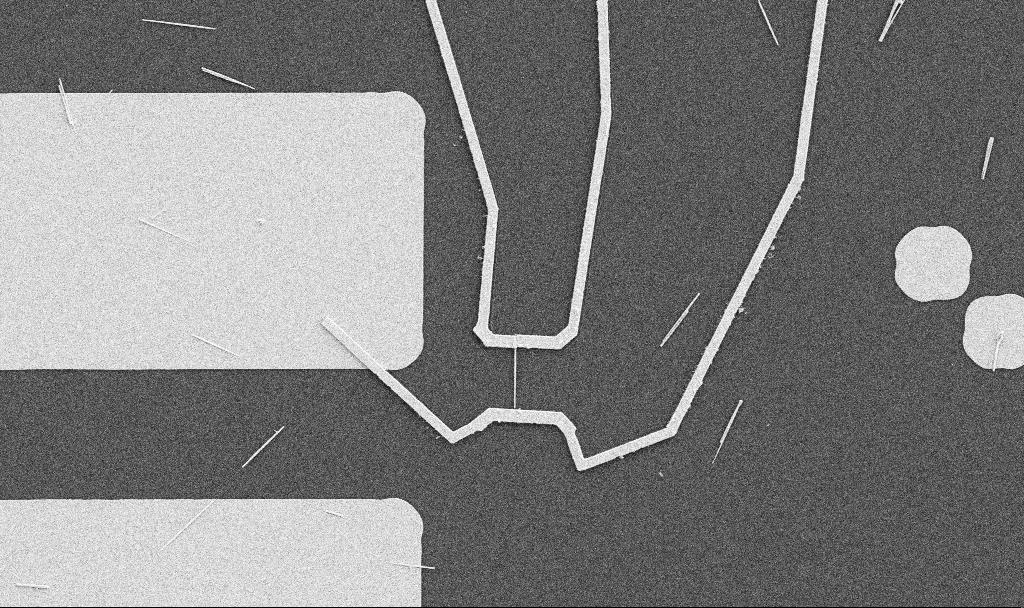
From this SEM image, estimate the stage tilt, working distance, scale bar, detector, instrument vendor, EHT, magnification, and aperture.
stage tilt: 0°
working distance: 10.7 mm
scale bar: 10000 nm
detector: SE2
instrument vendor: Zeiss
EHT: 5 kV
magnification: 5 K X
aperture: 30 µm